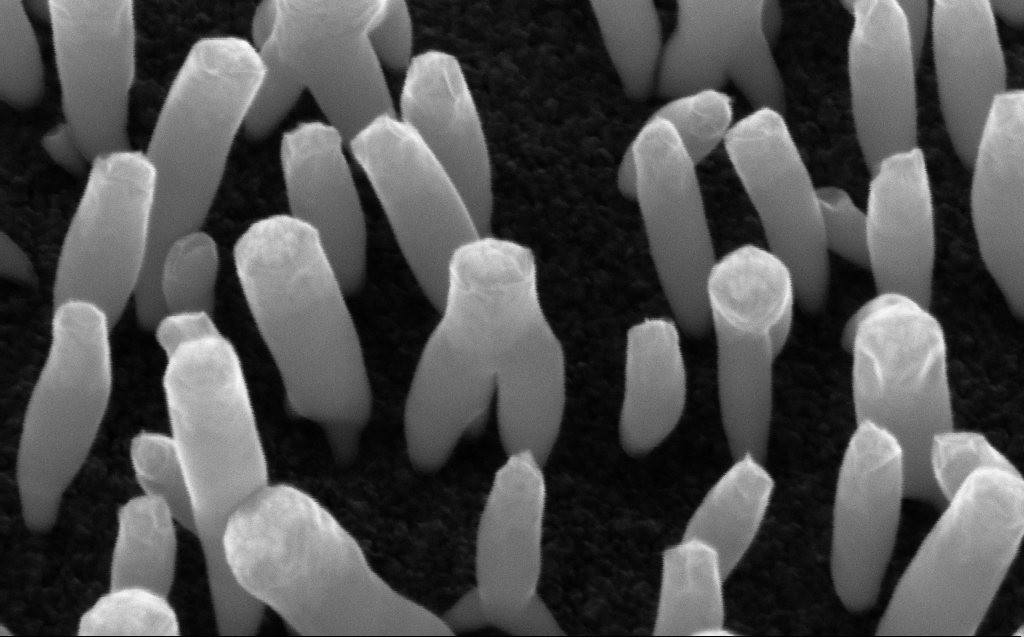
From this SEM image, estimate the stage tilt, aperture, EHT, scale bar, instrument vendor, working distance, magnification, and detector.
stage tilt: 45°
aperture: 30 µm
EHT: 10 kV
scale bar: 200 nm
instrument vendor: Zeiss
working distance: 5 mm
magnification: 241.16 K X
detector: InLens